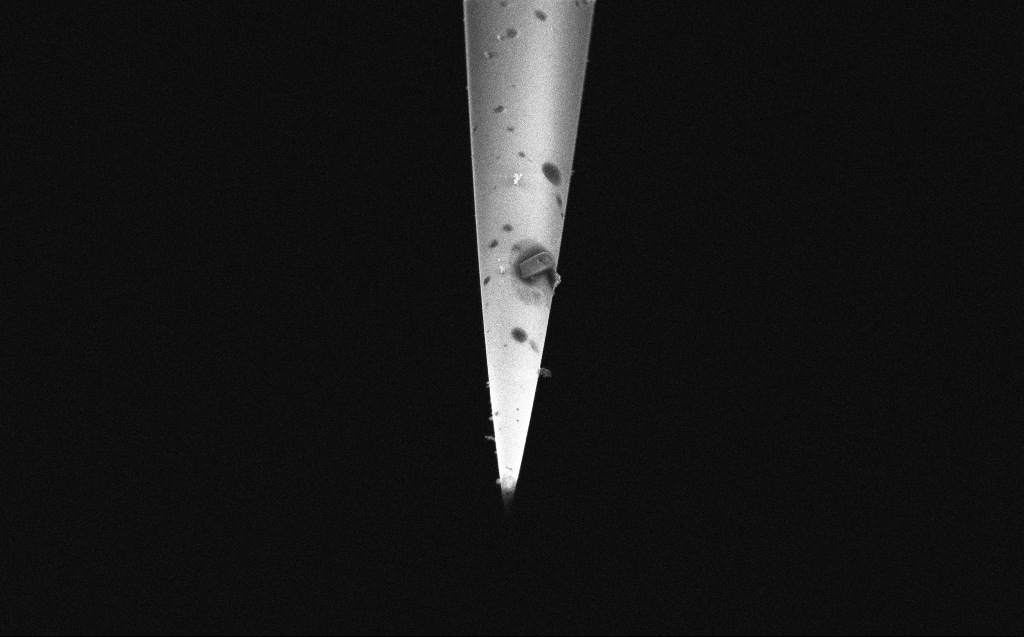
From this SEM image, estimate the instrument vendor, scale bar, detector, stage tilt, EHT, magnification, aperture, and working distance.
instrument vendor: Zeiss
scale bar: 2000 nm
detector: InLens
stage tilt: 45.1°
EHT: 3 kV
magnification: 15 K X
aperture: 20 µm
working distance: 6 mm